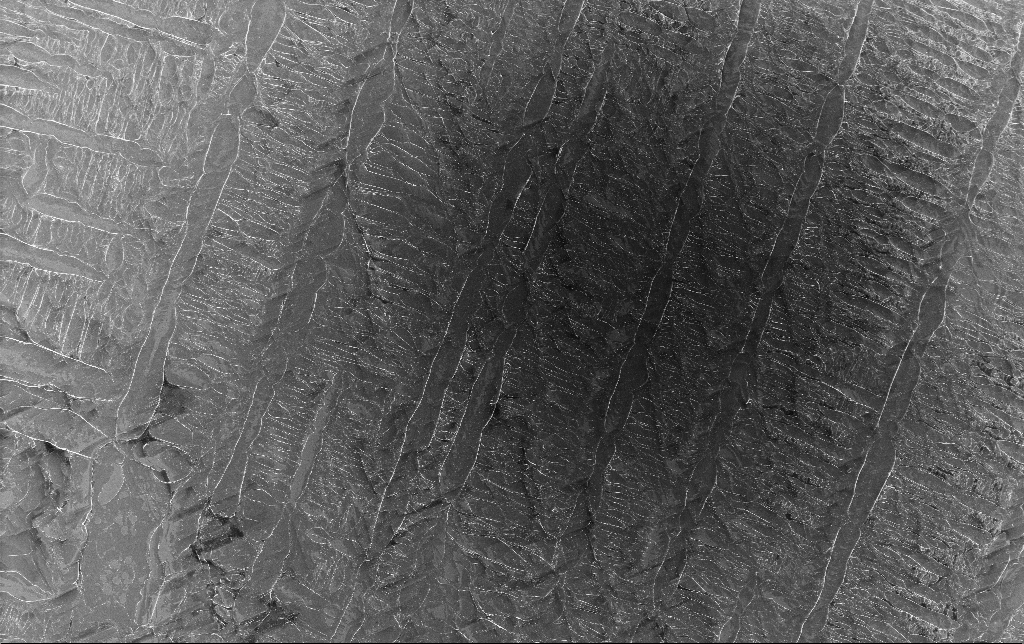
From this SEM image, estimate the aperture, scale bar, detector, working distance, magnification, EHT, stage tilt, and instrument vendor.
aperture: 30 µm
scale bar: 100000 nm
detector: InLens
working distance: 3.1 mm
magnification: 0.395 K X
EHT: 5 kV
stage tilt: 0°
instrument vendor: Zeiss